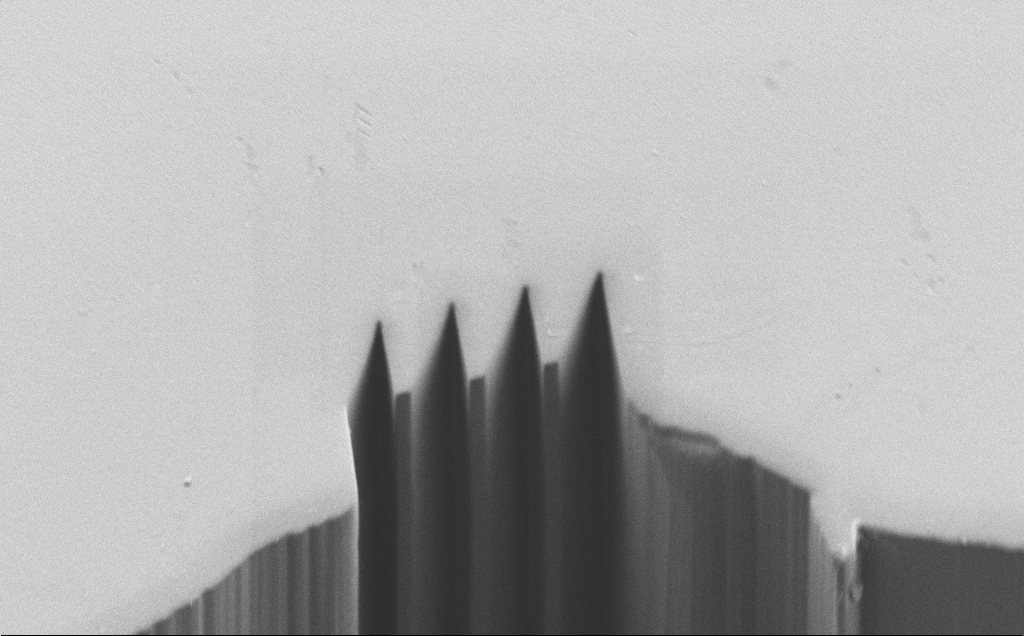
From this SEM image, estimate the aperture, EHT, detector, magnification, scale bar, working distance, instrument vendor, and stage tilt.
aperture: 30 µm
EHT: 1.2 kV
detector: SE2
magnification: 2.32 K X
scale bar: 20000 nm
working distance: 5 mm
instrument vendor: Zeiss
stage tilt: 40°